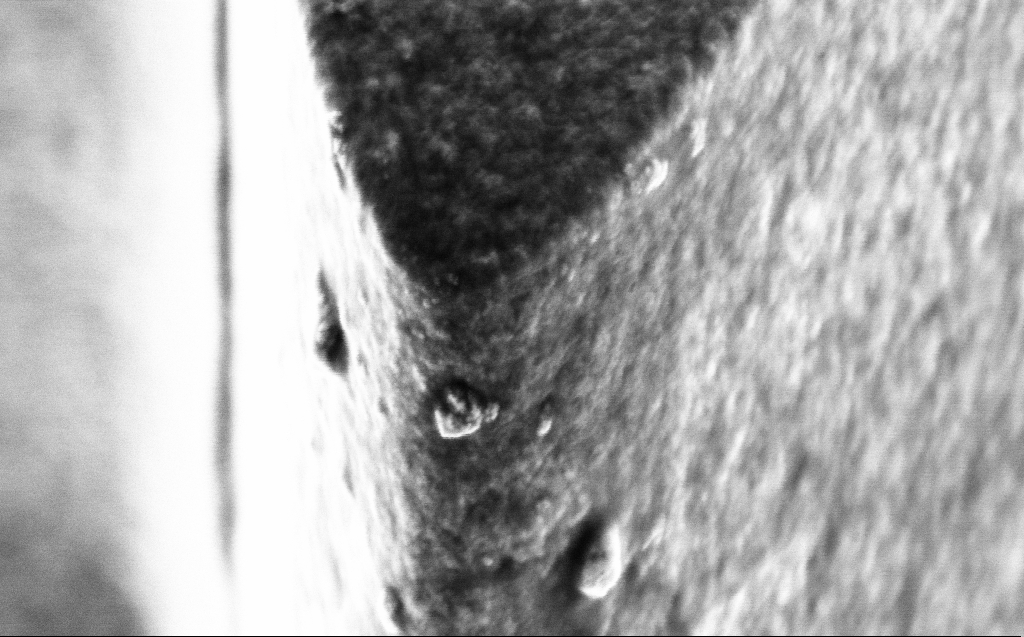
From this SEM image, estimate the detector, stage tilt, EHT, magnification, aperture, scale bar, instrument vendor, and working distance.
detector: InLens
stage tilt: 45°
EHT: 3 kV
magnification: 101.89 K X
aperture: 30 µm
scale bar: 200 nm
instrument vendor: Zeiss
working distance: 4 mm